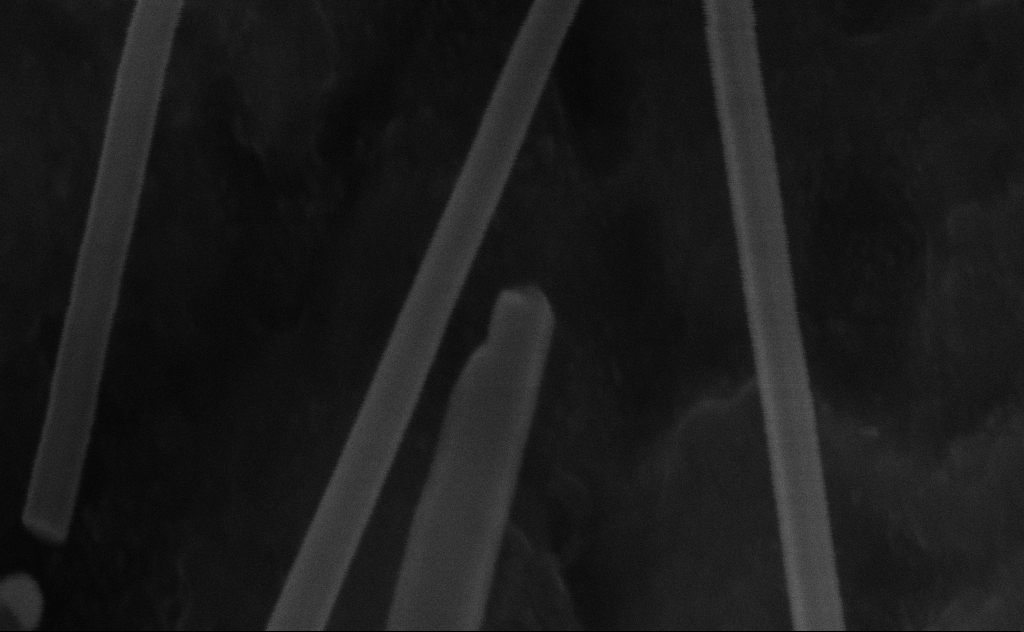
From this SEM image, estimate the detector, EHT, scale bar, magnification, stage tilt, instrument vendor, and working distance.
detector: InLens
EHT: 20 kV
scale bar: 100 nm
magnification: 434.91 K X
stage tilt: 0°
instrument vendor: Zeiss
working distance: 8 mm